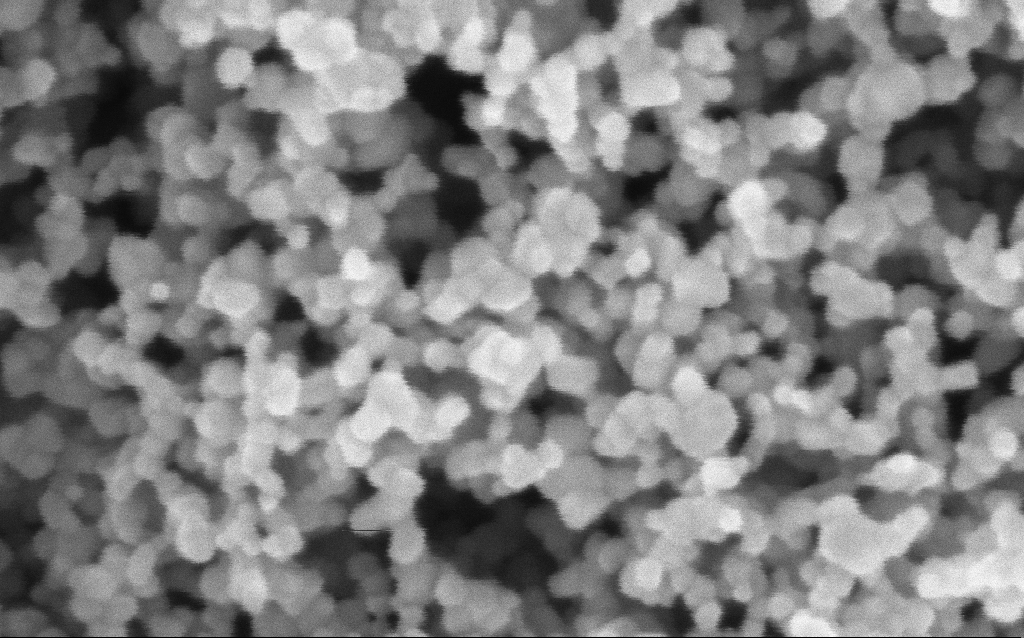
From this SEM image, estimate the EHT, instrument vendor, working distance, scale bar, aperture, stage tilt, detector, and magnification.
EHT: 3 kV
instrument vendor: Zeiss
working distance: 7.5 mm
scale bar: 100 nm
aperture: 30 µm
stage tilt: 0°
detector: InLens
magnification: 416 K X